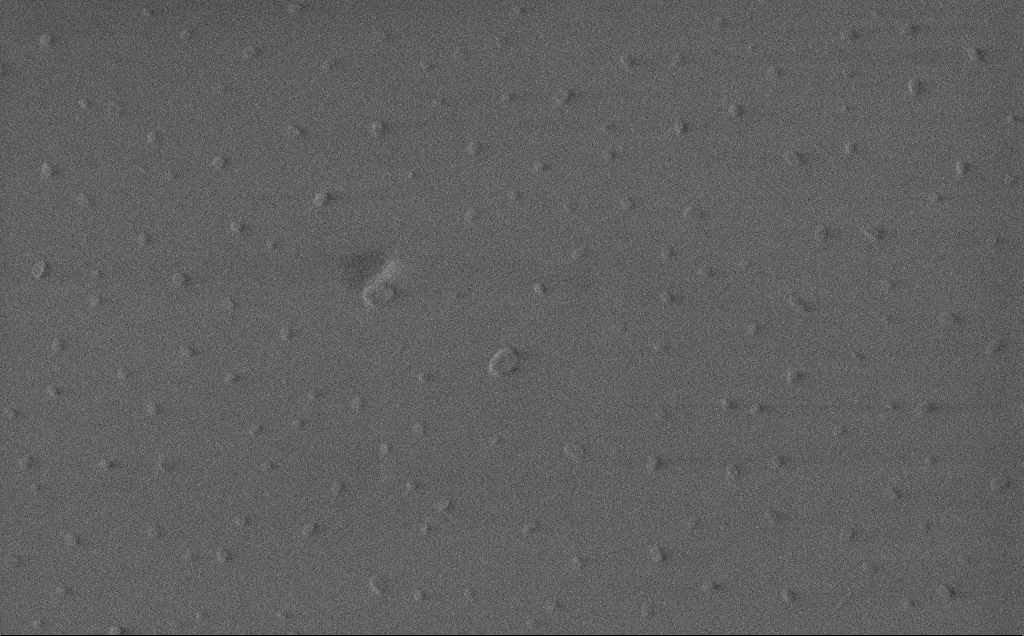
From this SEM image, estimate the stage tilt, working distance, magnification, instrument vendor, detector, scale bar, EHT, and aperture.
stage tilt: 0°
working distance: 3 mm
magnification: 59.97 K X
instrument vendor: Zeiss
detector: InLens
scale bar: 1000 nm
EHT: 1 kV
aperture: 30 µm